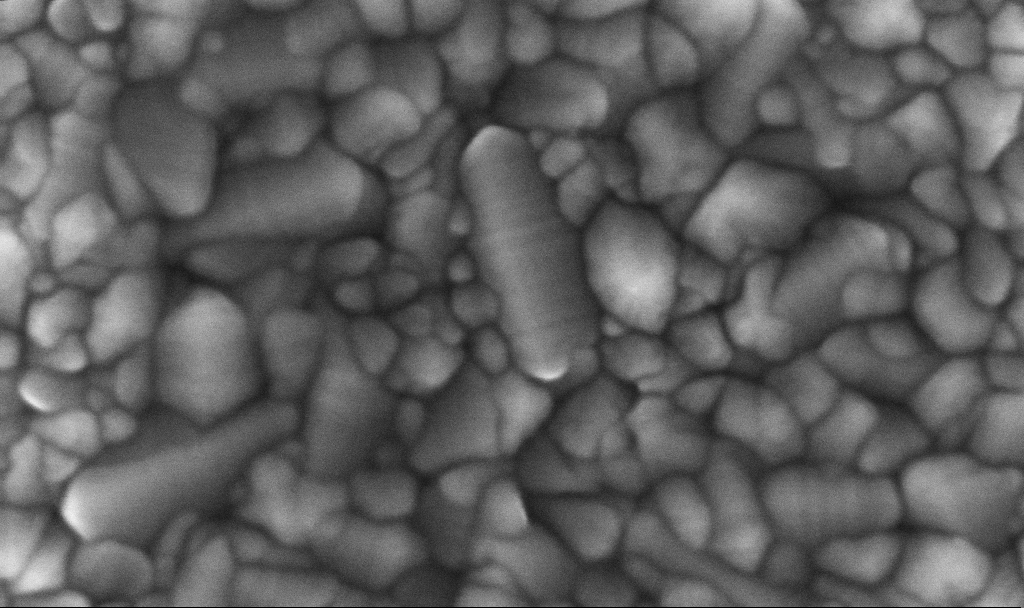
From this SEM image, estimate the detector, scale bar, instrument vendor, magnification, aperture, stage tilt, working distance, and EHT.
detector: InLens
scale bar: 100 nm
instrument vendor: Zeiss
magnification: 269.86 K X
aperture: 30 µm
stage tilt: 0°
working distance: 1.6 mm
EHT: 10 kV